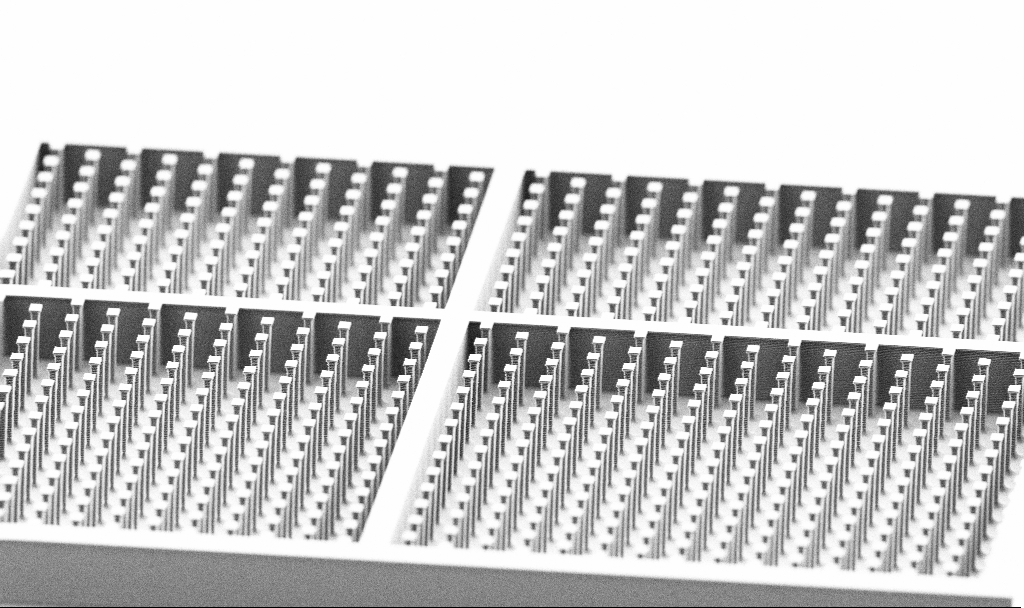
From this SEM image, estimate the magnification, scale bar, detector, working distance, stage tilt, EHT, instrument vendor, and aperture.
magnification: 1.43 K X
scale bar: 10000 nm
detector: SE2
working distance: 4.5 mm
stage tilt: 70°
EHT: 5 kV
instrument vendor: Zeiss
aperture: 30 µm